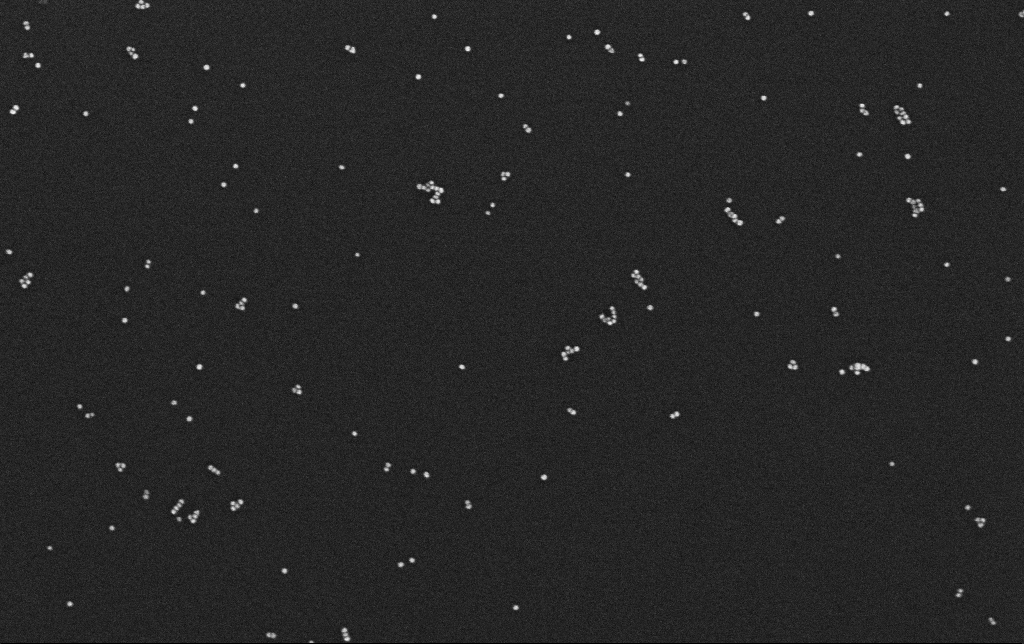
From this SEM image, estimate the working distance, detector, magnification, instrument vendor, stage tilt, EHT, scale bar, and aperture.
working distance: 3.1 mm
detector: InLens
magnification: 120.18 K X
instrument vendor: Zeiss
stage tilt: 0°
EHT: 10 kV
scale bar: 200 nm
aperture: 30 µm